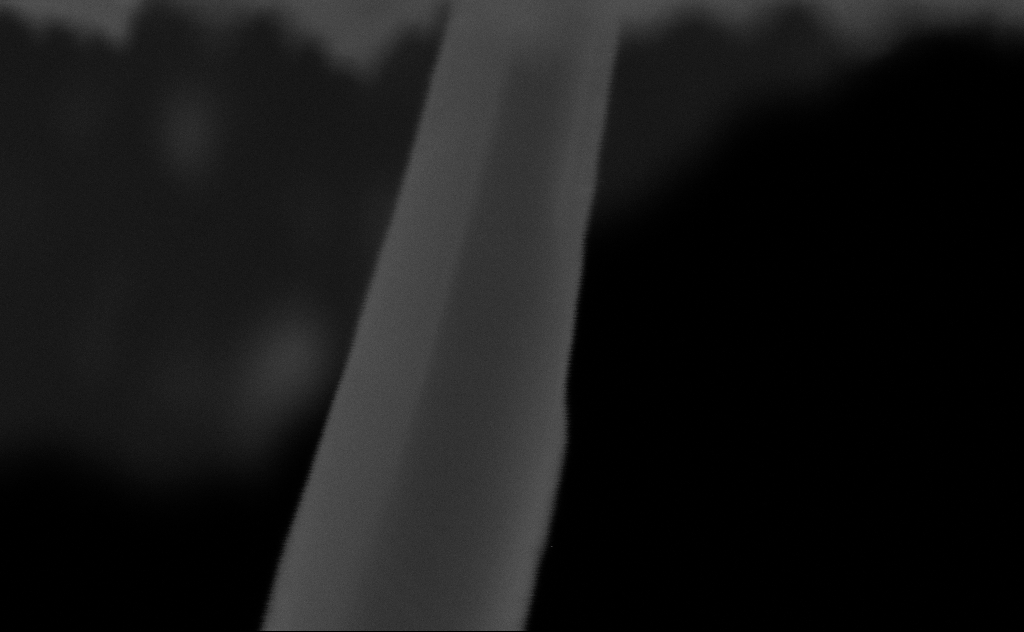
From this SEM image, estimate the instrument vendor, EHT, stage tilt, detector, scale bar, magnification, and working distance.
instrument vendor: Zeiss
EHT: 20 kV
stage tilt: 0°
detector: SE2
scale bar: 20 nm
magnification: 761.93 K X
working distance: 8 mm